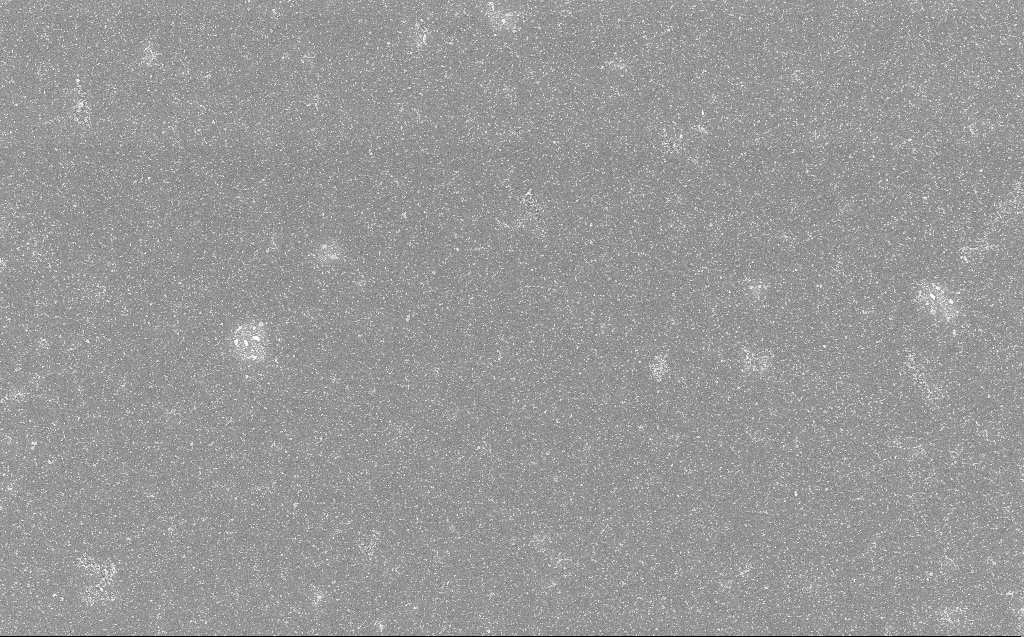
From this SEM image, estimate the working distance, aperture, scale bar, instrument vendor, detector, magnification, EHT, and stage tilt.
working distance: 3 mm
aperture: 30 µm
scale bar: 10000 nm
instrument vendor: Zeiss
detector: InLens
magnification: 5 K X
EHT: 10 kV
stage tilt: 0°